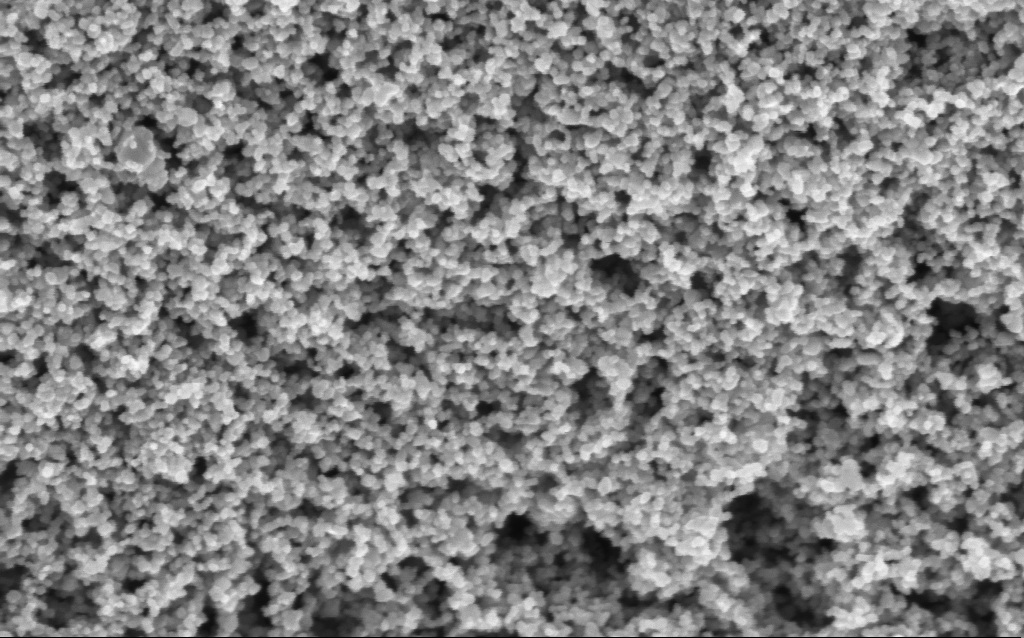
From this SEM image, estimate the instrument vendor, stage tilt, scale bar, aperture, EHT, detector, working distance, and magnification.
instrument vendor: Zeiss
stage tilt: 0°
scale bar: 100 nm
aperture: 30 µm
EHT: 3 kV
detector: InLens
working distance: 7.6 mm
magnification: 130 K X